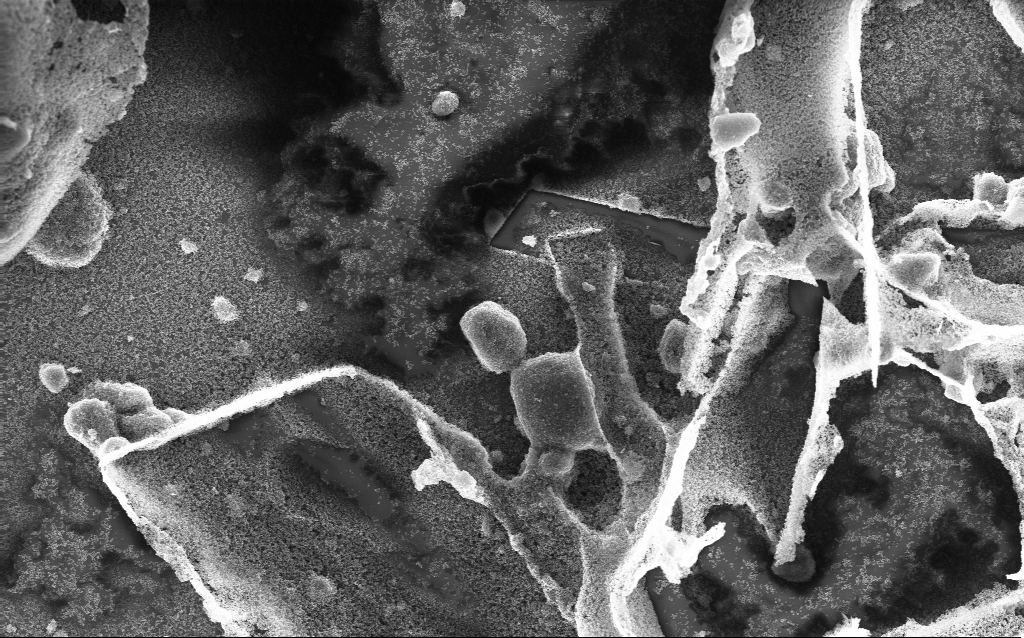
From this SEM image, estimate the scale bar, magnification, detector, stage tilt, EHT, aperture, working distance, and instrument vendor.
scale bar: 10000 nm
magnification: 5.83 K X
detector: InLens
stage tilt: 0°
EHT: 10 kV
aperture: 30 µm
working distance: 2.4 mm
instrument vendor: Zeiss